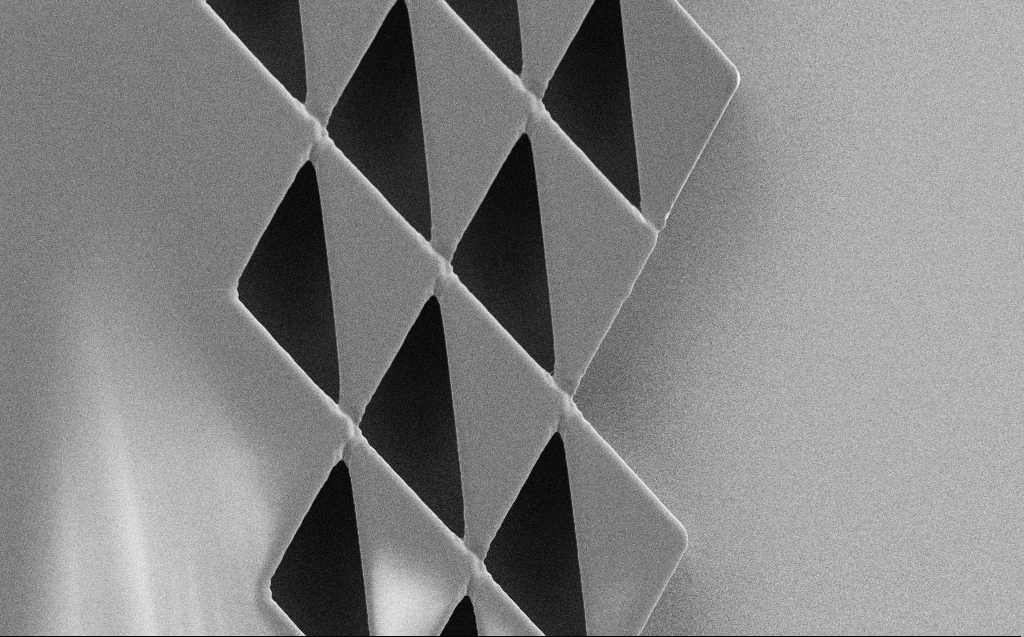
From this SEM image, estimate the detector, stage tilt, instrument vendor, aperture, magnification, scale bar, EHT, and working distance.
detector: SE2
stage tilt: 0°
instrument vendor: Zeiss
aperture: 30 µm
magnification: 1.11 K X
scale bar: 20000 nm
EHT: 1 kV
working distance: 6 mm